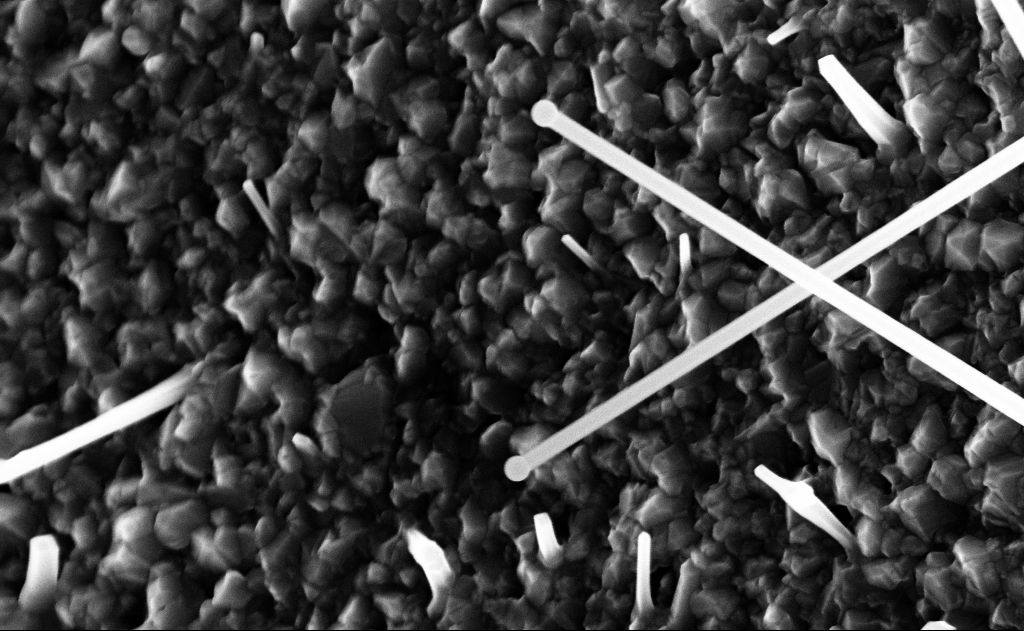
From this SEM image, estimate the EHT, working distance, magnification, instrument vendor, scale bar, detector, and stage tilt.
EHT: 10 kV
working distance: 9 mm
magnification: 60 K X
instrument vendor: Zeiss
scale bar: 1000 nm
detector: InLens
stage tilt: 0°